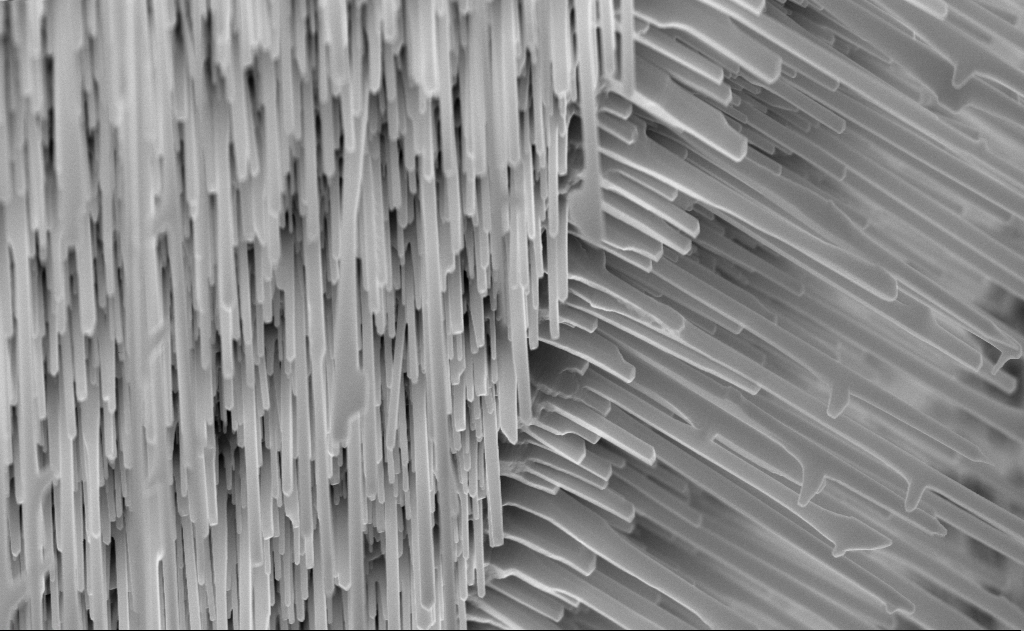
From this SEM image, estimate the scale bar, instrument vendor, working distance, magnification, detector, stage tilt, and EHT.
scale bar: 1000 nm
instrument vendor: Zeiss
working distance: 6 mm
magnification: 40 K X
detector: InLens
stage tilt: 0°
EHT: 10 kV